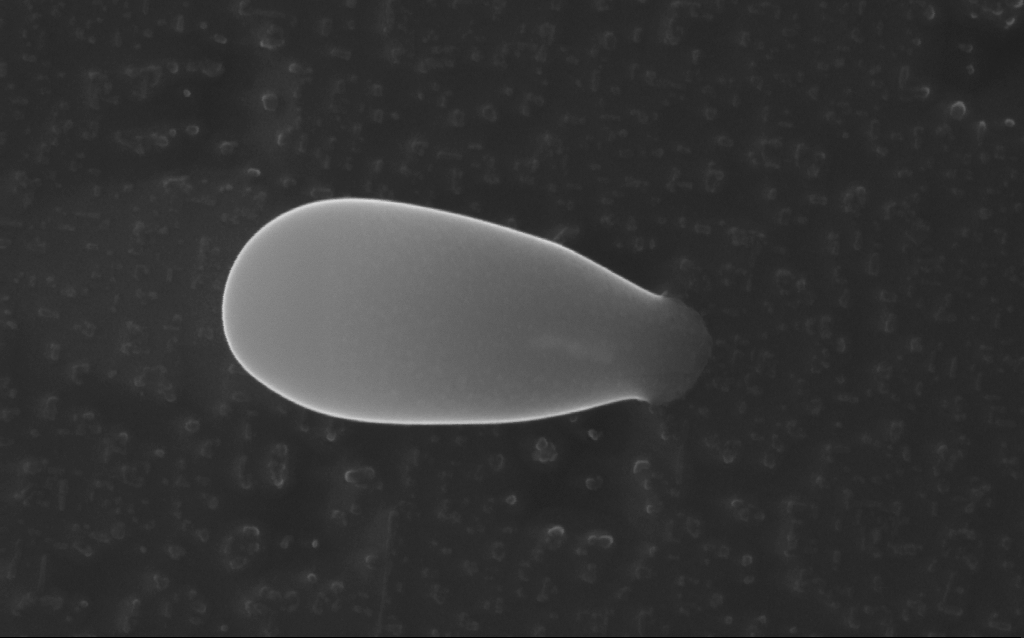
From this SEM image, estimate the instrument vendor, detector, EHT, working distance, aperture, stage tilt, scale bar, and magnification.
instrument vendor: Zeiss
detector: InLens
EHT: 10 kV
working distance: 5 mm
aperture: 30 µm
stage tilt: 0°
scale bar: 200 nm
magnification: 171.95 K X